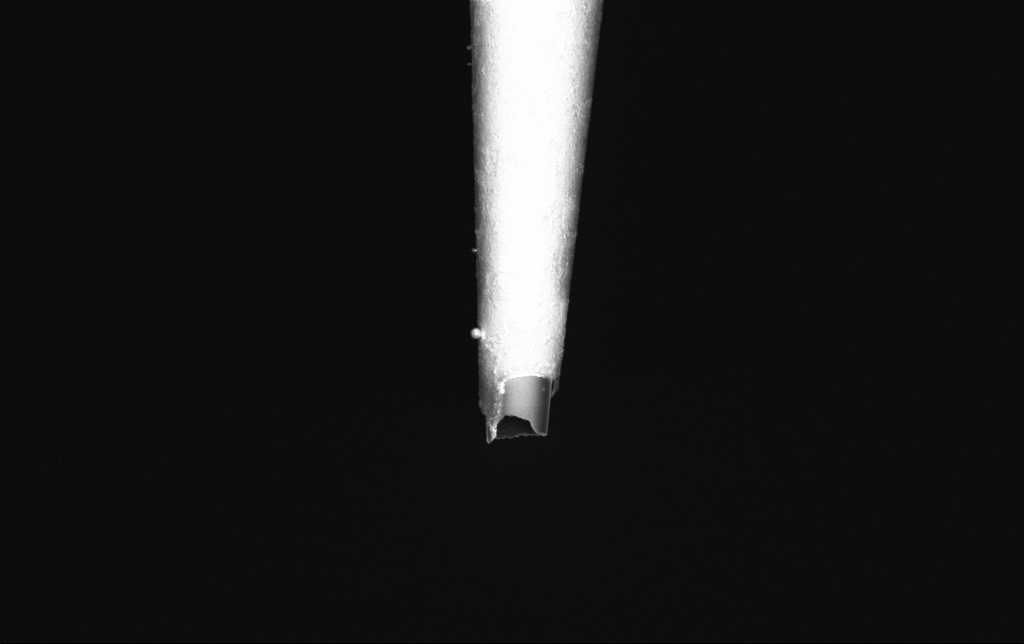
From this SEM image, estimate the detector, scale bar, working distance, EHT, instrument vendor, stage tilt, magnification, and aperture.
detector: InLens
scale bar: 2000 nm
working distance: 6.1 mm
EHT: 2 kV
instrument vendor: Zeiss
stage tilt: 0°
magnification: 10 K X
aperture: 30 µm